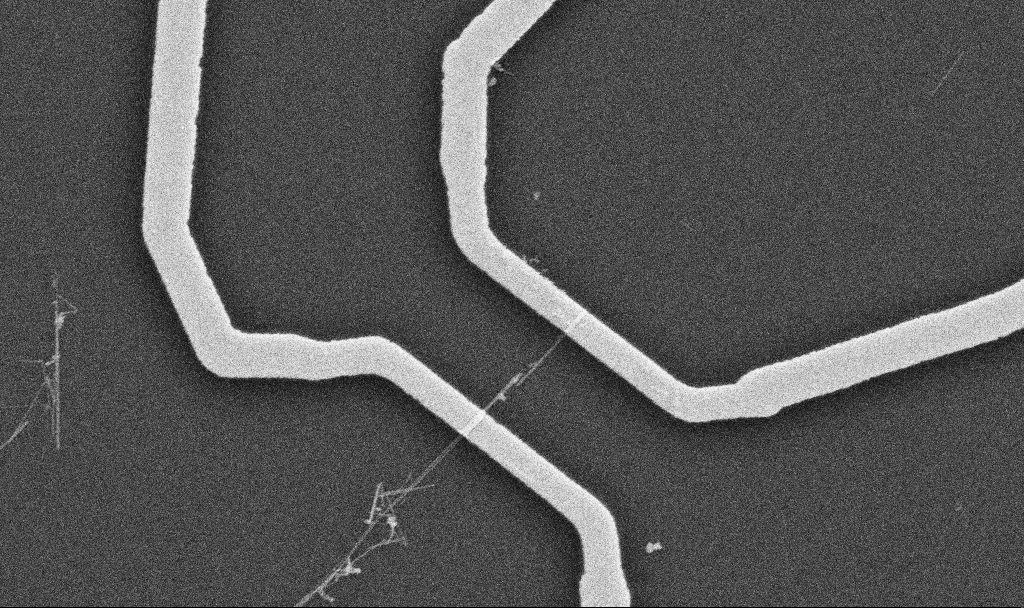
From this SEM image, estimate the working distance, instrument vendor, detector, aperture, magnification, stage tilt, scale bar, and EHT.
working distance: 10.7 mm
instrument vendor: Zeiss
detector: SE2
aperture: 30 µm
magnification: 20 K X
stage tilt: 0°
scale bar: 1000 nm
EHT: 10 kV